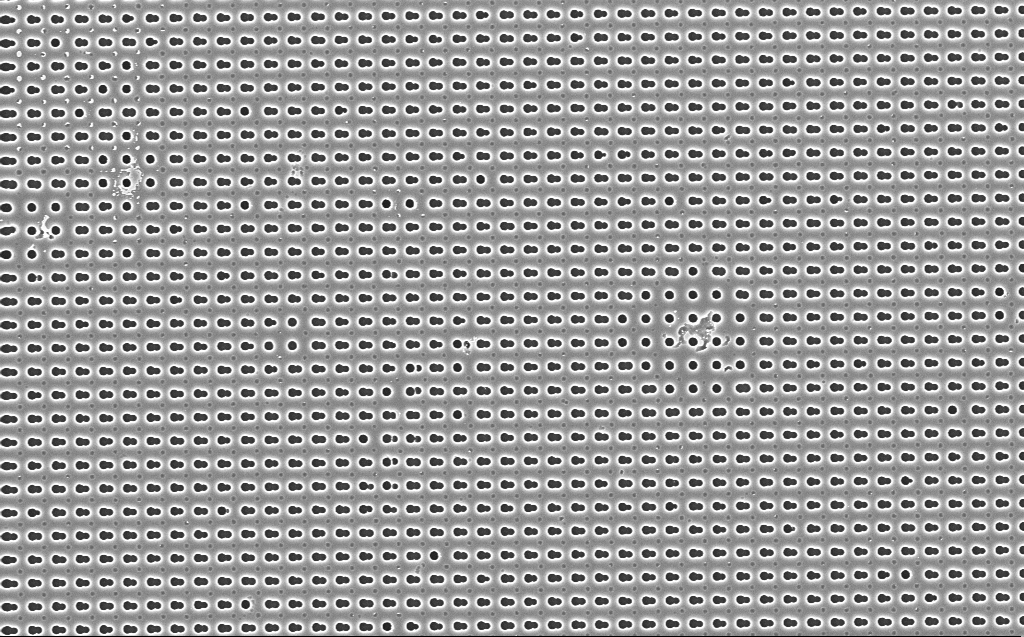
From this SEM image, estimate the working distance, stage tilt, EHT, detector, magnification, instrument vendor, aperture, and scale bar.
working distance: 4 mm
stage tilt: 0°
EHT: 5 kV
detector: InLens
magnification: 8.32 K X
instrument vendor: Zeiss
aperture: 30 µm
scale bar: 2000 nm